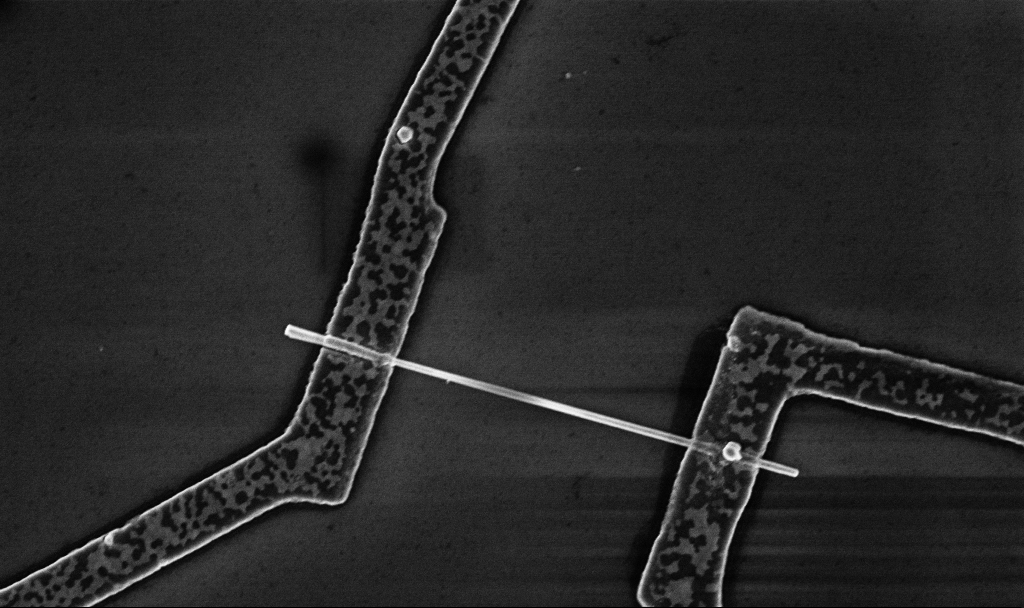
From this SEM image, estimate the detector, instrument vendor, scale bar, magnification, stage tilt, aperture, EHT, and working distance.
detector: InLens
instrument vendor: Zeiss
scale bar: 1000 nm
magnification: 30 K X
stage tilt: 0°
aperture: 30 µm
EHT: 5 kV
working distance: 8.7 mm